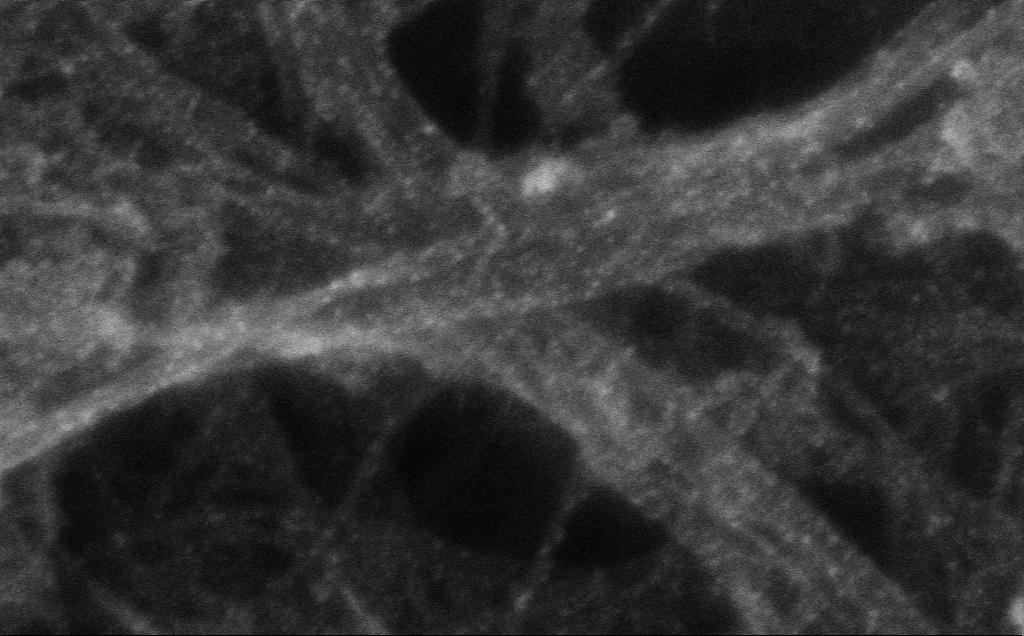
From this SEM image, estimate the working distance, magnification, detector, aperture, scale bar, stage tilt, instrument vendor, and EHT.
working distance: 3 mm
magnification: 609.25 K X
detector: InLens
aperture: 30 µm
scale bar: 100 nm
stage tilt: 0°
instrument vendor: Zeiss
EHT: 10 kV